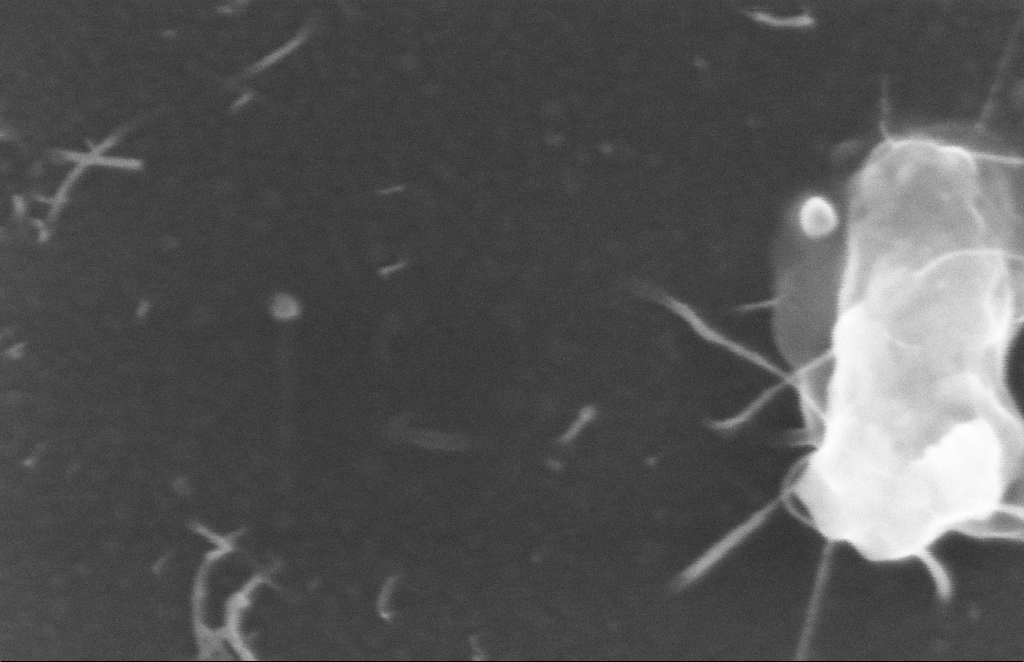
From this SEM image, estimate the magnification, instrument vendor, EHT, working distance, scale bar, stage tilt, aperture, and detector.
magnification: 399.53 K X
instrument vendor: Zeiss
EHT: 5 kV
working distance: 8 mm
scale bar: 100 nm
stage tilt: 0°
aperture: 20 µm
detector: InLens